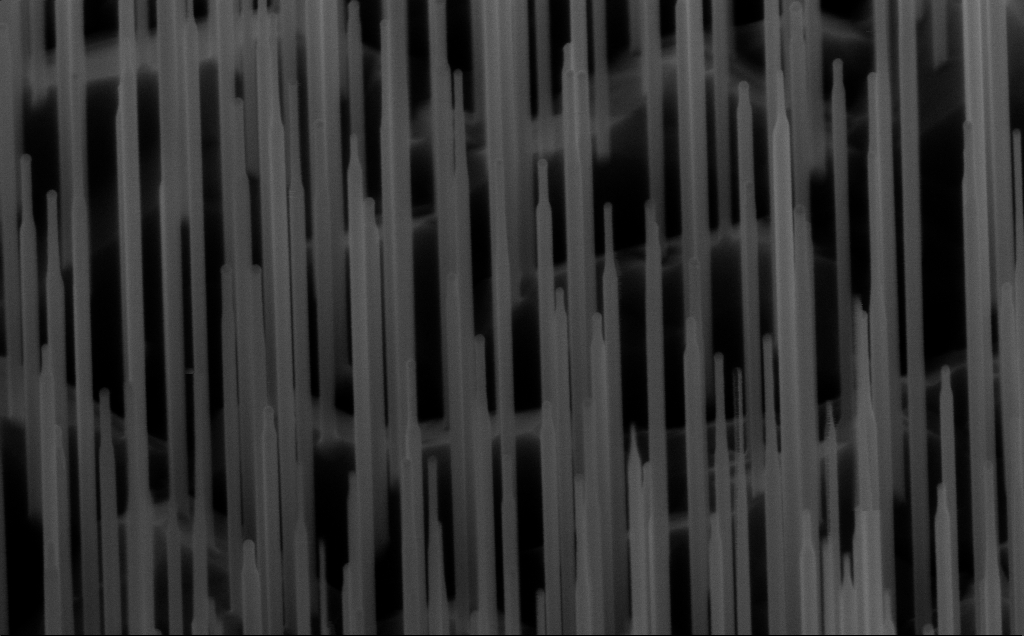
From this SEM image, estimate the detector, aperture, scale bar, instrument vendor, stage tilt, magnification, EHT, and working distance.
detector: InLens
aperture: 30 µm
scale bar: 200 nm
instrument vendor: Zeiss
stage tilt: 45°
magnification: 80 K X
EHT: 10 kV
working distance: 6 mm